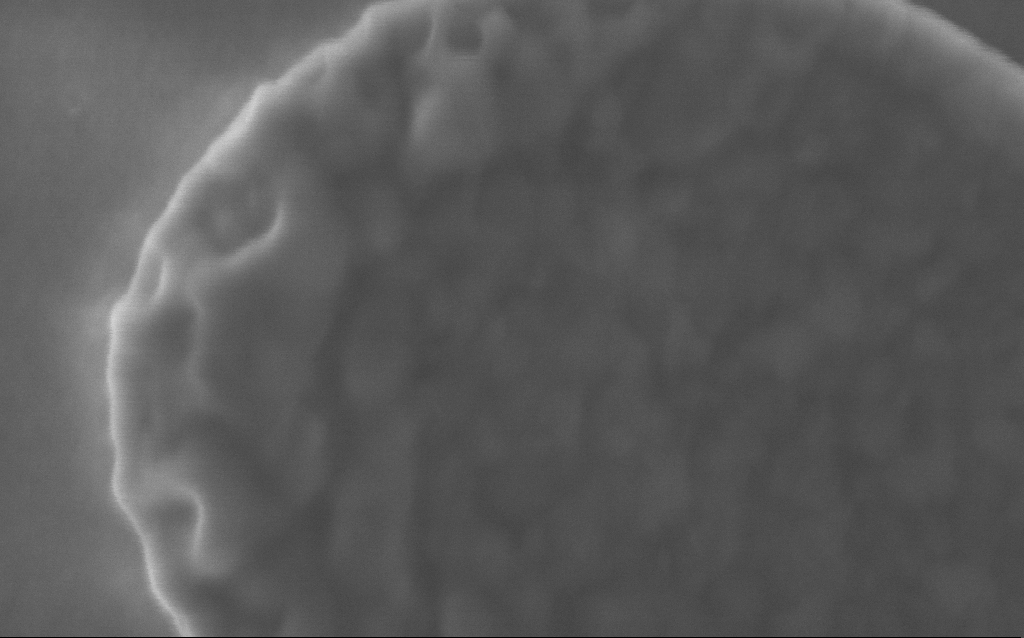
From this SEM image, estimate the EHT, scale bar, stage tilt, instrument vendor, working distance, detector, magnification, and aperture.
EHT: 5 kV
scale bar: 200 nm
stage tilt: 0°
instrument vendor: Zeiss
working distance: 4 mm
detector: InLens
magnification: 234 K X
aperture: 30 µm